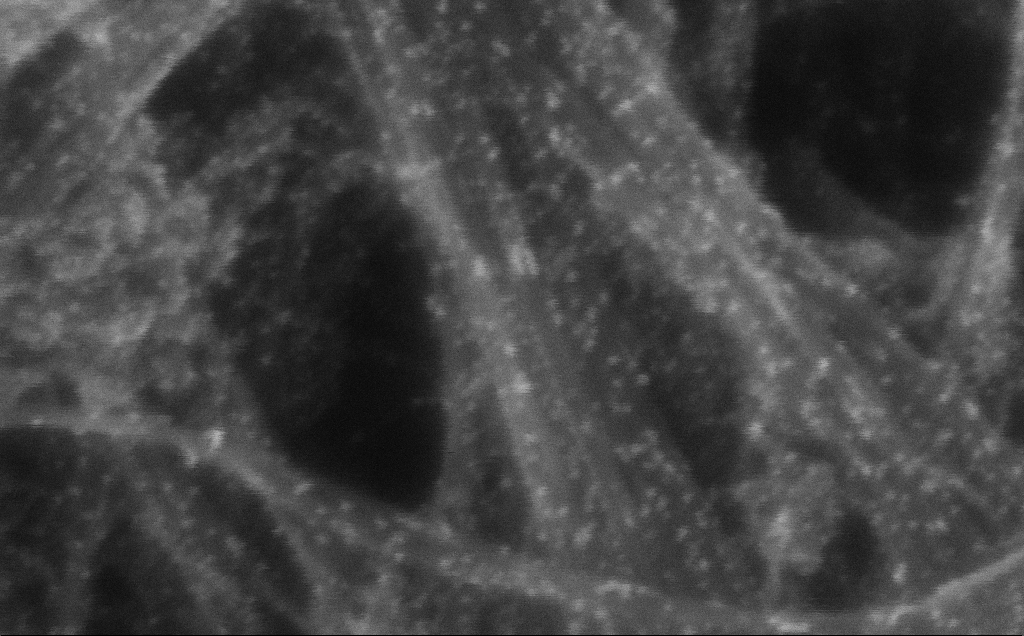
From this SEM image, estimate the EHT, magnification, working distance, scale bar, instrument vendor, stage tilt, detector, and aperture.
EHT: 10 kV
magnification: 790.09 K X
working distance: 3 mm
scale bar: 20 nm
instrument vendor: Zeiss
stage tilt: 0°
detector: InLens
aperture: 30 µm